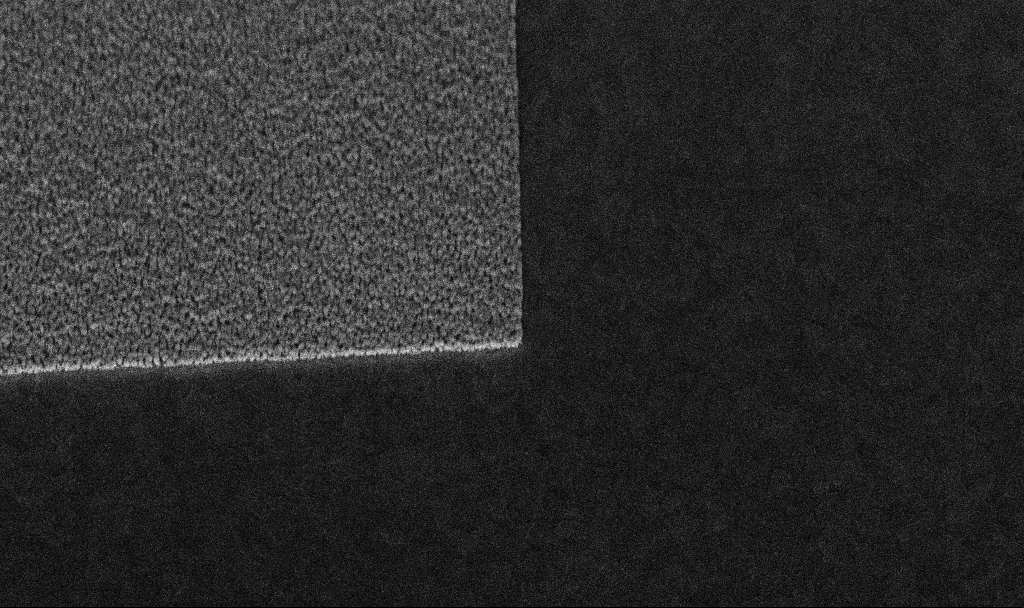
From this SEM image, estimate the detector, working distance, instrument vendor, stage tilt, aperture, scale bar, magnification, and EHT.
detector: InLens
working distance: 6.4 mm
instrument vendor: Zeiss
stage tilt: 45°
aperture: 30 µm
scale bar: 2000 nm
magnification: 28.27 K X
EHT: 5 kV